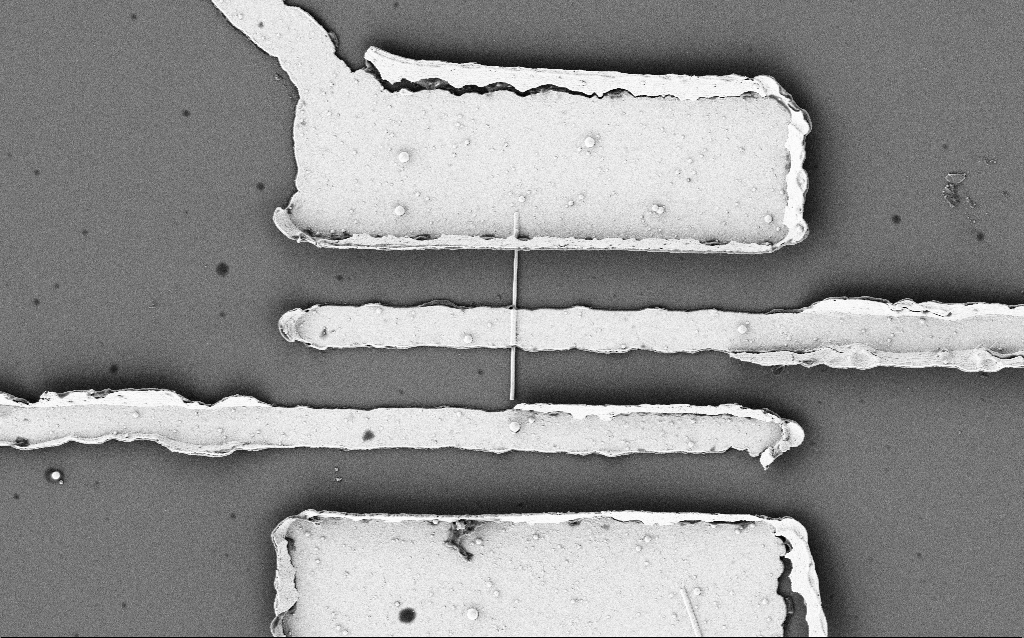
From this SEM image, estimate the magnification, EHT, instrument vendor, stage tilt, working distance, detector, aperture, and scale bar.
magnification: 6.3 K X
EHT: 2 kV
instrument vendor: Zeiss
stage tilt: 0°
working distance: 7.6 mm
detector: SE2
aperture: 30 µm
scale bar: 10000 nm